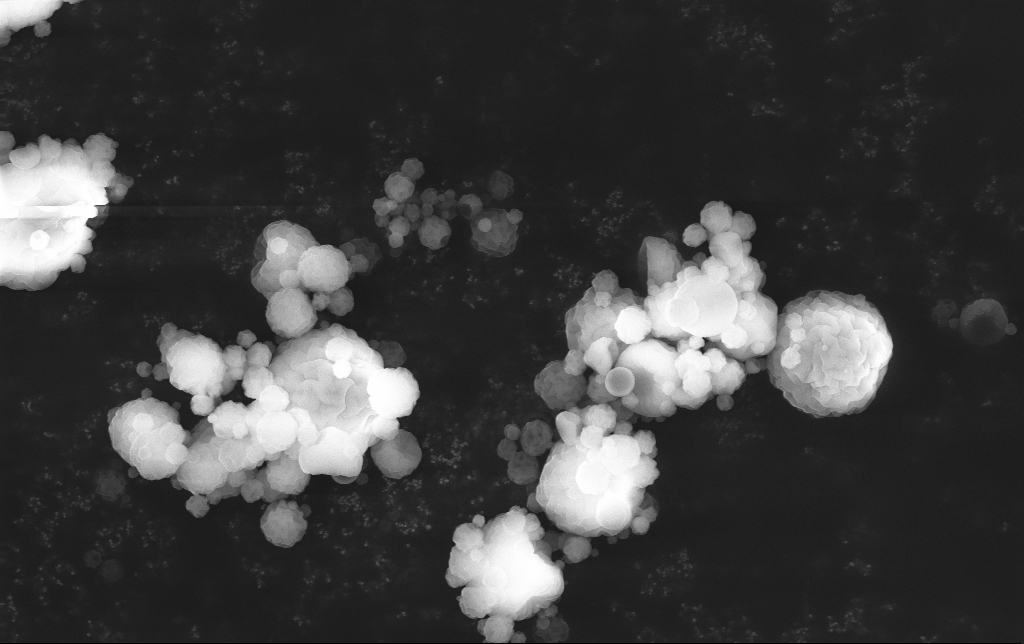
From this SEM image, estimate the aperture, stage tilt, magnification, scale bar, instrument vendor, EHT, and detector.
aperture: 30 µm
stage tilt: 0°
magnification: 9.81 K X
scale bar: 2000 nm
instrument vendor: Zeiss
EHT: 15 kV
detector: InLens